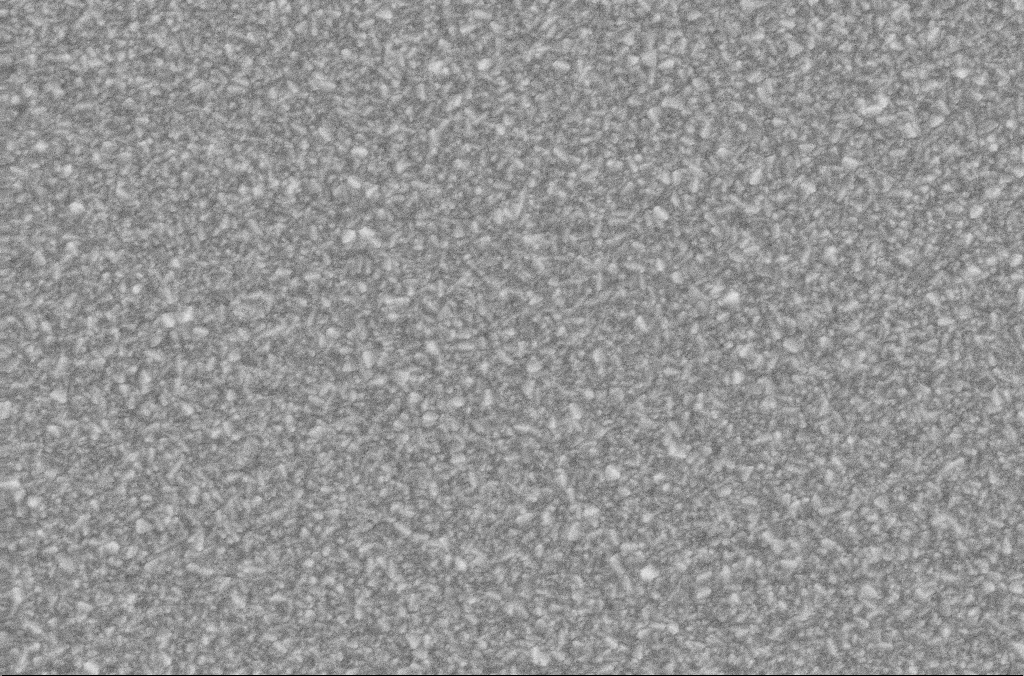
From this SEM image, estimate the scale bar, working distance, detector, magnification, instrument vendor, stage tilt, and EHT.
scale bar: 2000 nm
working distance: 1.9 mm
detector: SE2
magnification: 15 K X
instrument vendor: Zeiss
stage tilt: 0°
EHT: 20 kV